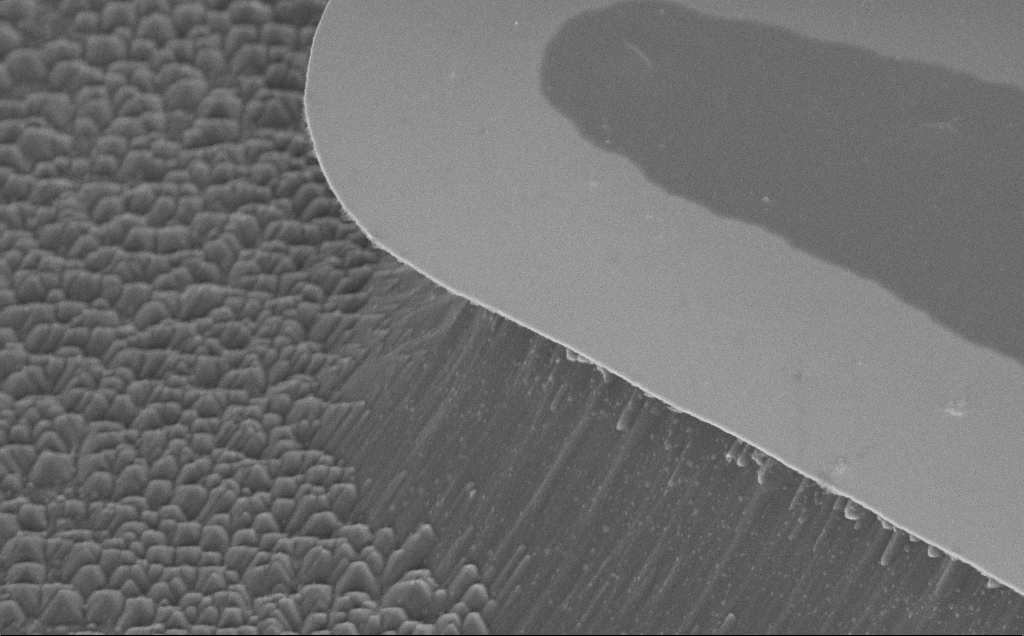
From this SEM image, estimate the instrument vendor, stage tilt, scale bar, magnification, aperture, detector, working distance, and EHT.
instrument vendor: Zeiss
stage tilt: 45°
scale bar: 2000 nm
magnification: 12.16 K X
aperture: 30 µm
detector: InLens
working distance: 13 mm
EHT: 5 kV